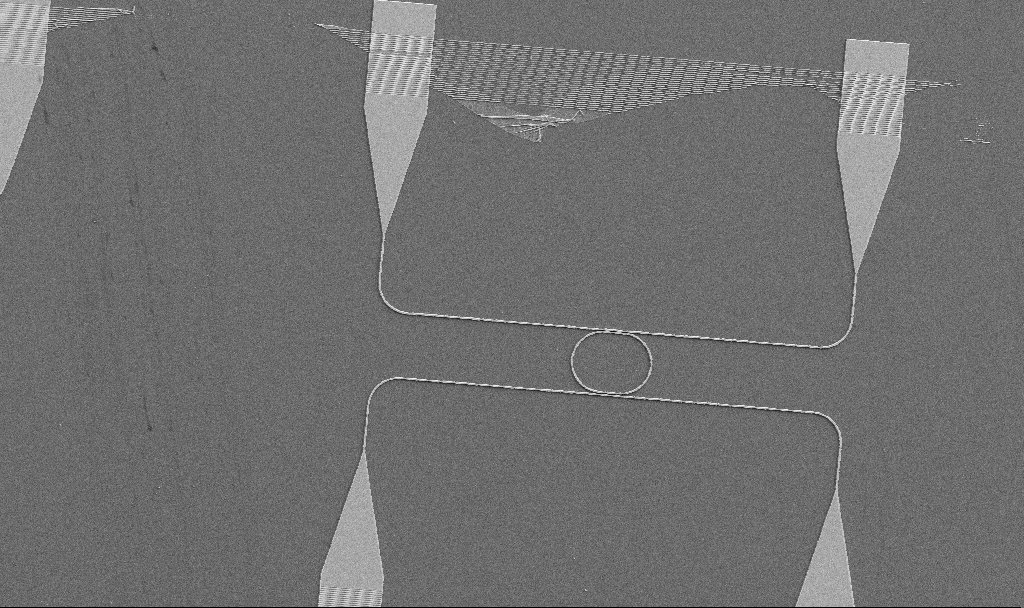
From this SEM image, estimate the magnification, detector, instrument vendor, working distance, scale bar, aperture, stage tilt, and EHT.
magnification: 1.15 K X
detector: SE2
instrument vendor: Zeiss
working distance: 7.4 mm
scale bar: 20000 nm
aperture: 30 µm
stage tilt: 0°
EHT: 5 kV